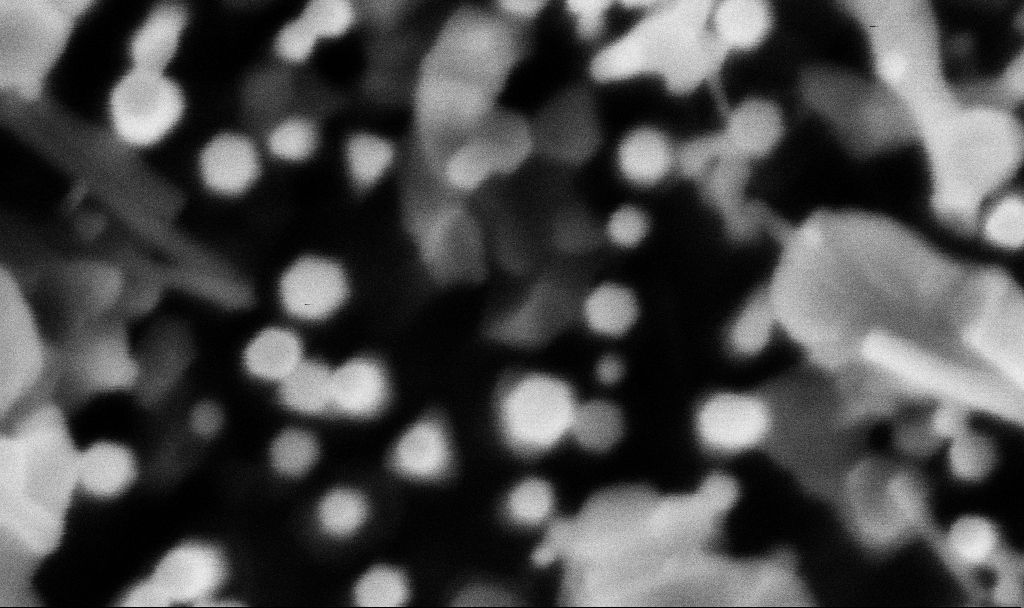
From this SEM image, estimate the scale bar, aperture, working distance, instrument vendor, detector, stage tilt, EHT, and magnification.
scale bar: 100 nm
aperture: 30 µm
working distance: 3.1 mm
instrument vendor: Zeiss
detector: InLens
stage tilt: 0°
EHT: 3 kV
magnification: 462.21 K X